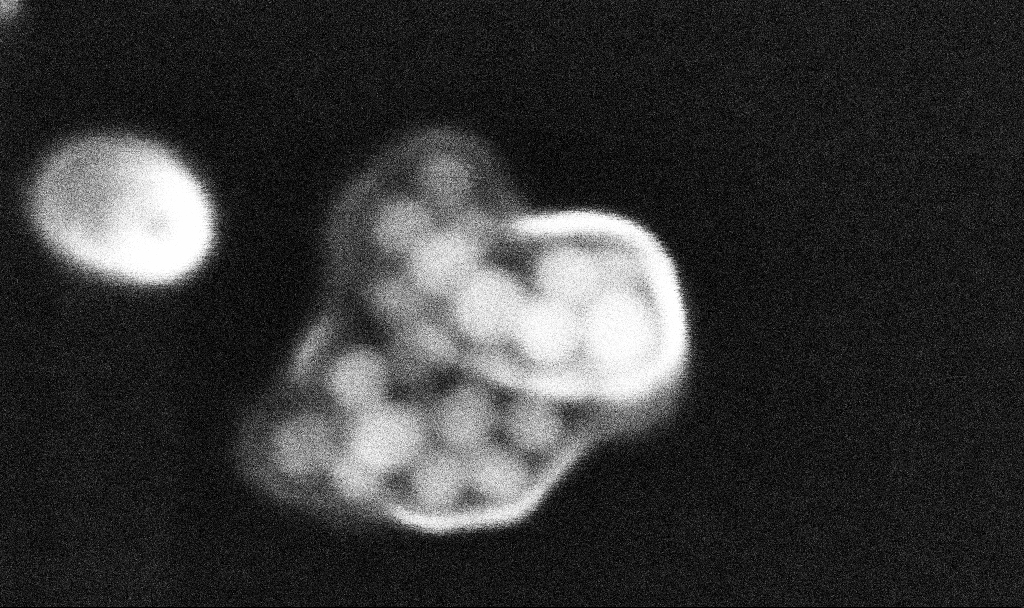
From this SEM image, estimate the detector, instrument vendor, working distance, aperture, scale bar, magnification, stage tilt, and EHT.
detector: InLens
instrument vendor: Zeiss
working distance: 3.3 mm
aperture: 30 µm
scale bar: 20 nm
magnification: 1192.45 K X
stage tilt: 0°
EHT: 10 kV